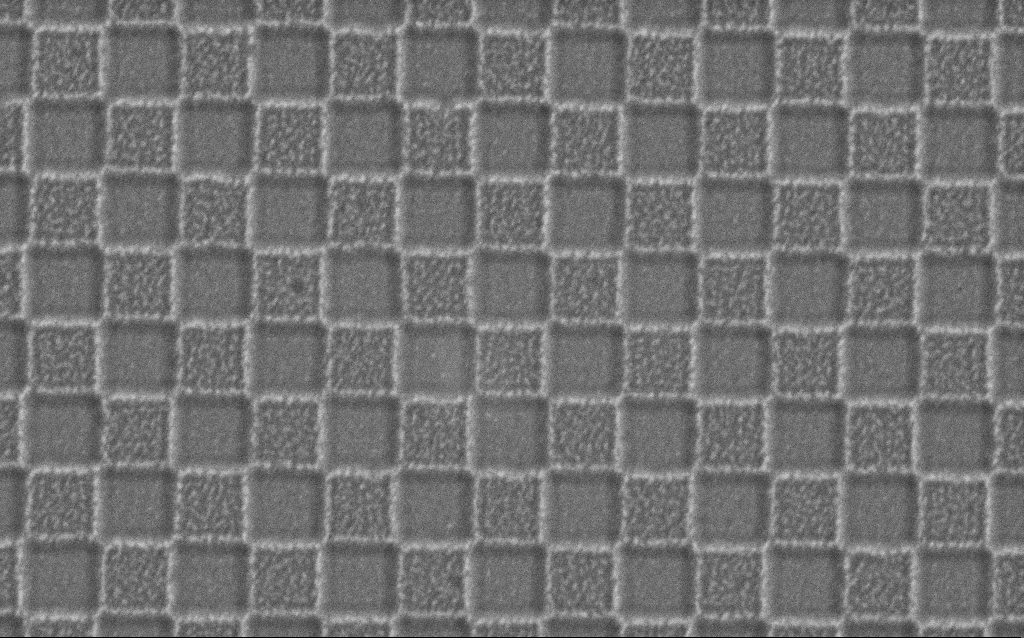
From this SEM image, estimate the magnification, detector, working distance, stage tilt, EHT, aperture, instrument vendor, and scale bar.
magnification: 54.33 K X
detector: SE2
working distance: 6 mm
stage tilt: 0°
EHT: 1.5 kV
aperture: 30 µm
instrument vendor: Zeiss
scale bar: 1000 nm